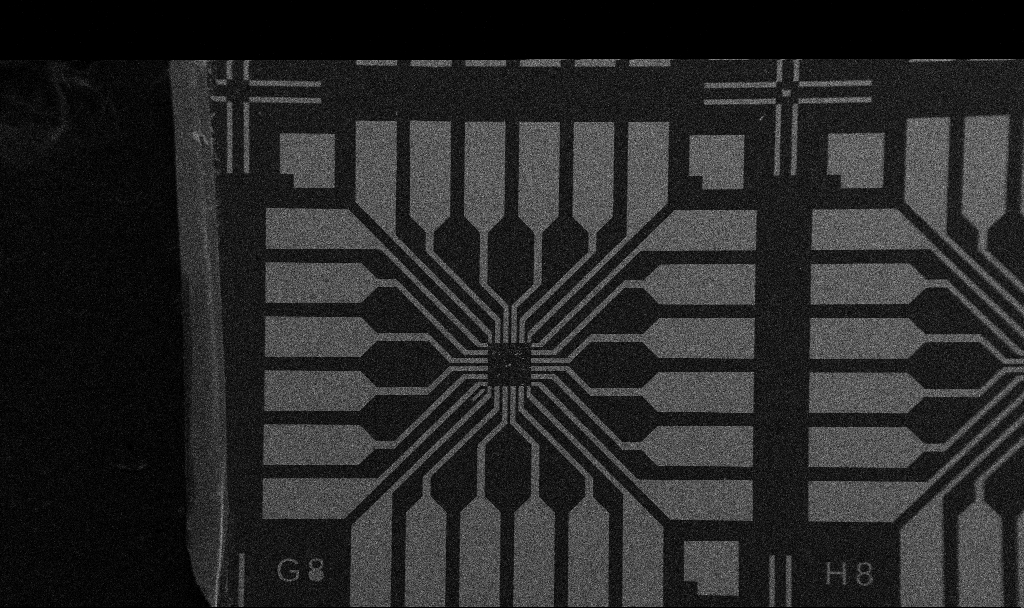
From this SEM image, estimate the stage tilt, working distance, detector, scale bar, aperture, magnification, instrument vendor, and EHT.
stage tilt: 0°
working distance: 10.7 mm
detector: SE2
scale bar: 200000 nm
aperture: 30 µm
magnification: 0.1 K X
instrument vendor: Zeiss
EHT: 5 kV